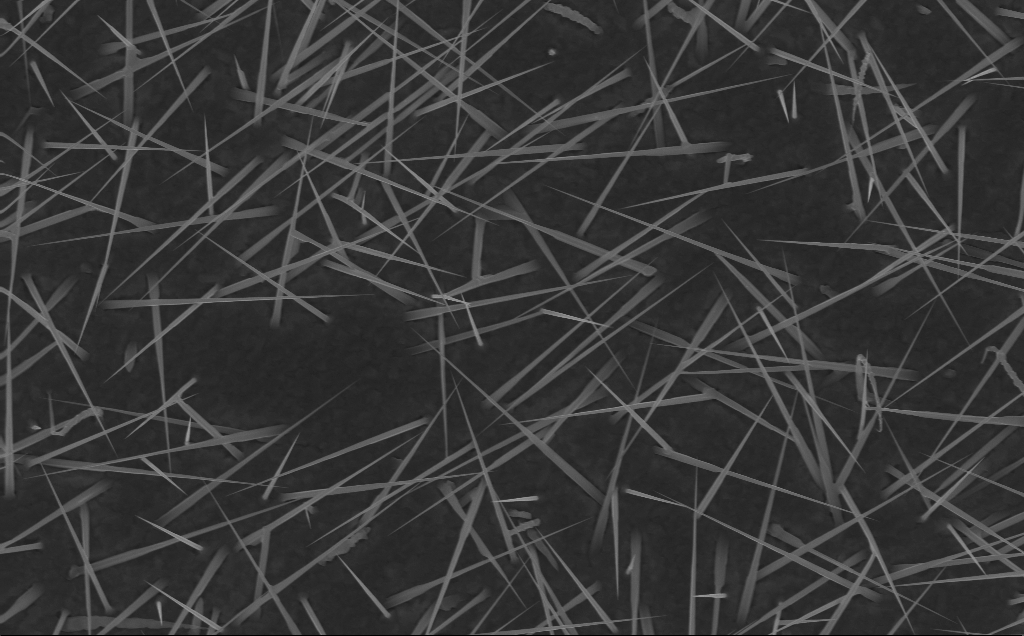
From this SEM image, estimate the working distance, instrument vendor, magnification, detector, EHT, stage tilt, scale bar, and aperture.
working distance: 6 mm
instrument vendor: Zeiss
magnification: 20 K X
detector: InLens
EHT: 10 kV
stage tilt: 0°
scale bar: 1000 nm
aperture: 30 µm